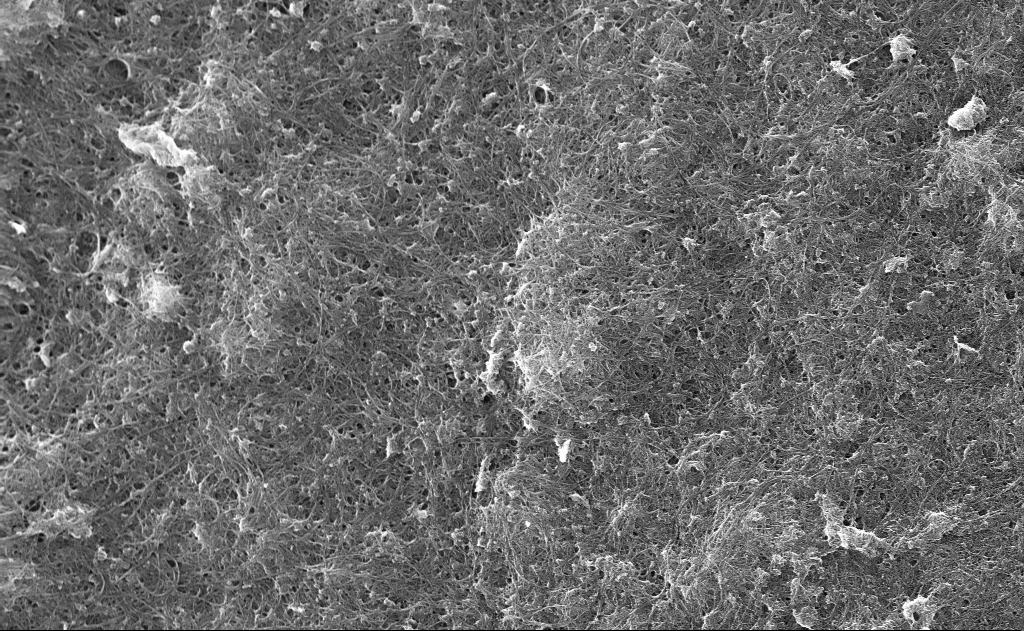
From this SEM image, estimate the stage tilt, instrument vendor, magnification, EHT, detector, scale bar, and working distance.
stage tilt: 0°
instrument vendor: Zeiss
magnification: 8.95 K X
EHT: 10 kV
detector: InLens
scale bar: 2000 nm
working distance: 6 mm